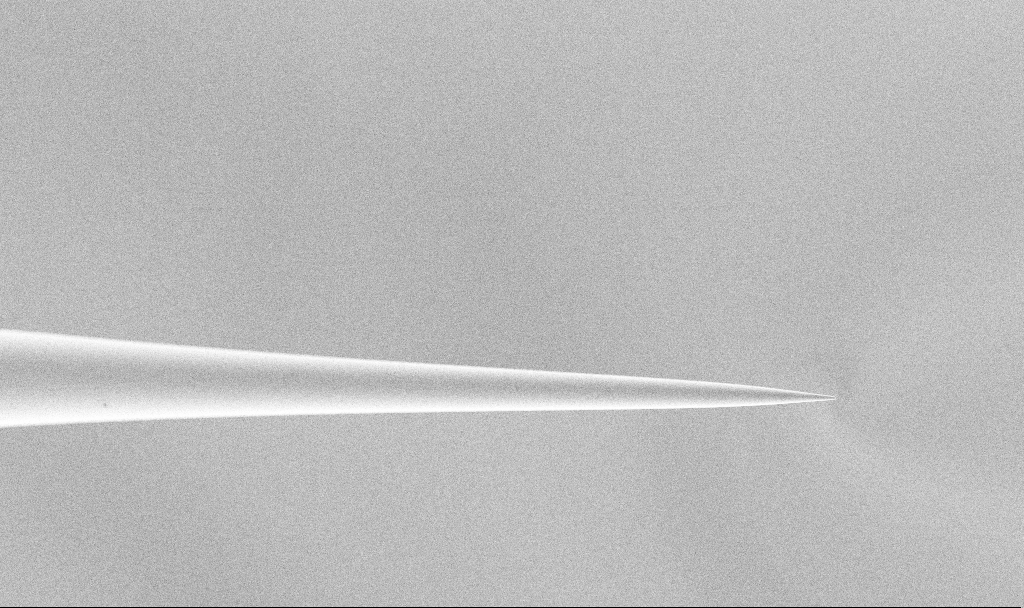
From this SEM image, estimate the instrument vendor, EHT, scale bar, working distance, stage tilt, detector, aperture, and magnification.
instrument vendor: Zeiss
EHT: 1.5 kV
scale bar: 10000 nm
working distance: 3.2 mm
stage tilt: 45°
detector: SE2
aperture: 30 µm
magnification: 1.34 K X